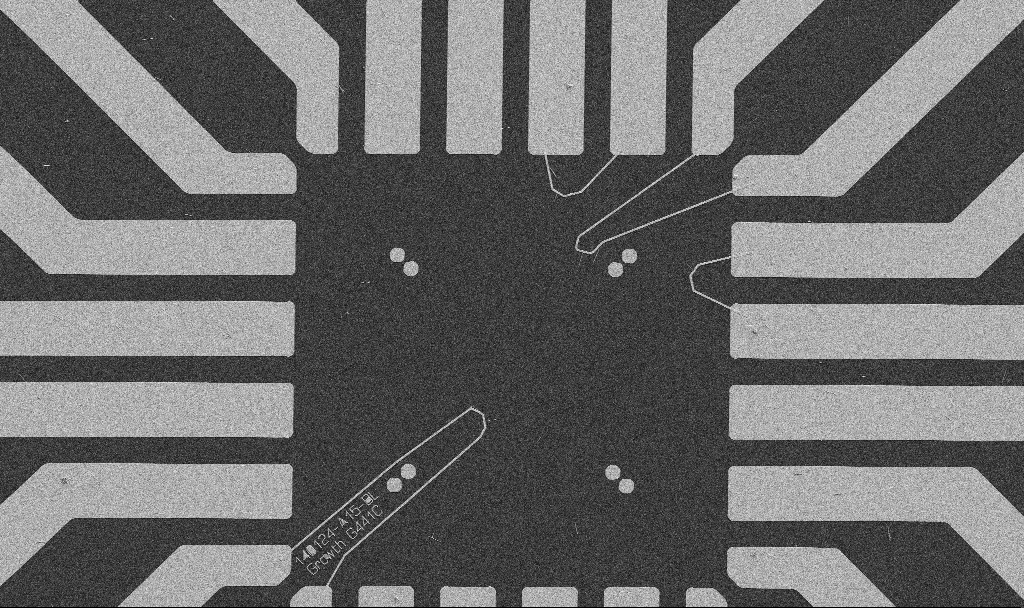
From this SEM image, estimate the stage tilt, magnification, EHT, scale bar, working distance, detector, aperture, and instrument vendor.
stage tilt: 0°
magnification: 1 K X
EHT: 5 kV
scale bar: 20000 nm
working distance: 10.7 mm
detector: SE2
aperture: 30 µm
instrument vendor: Zeiss